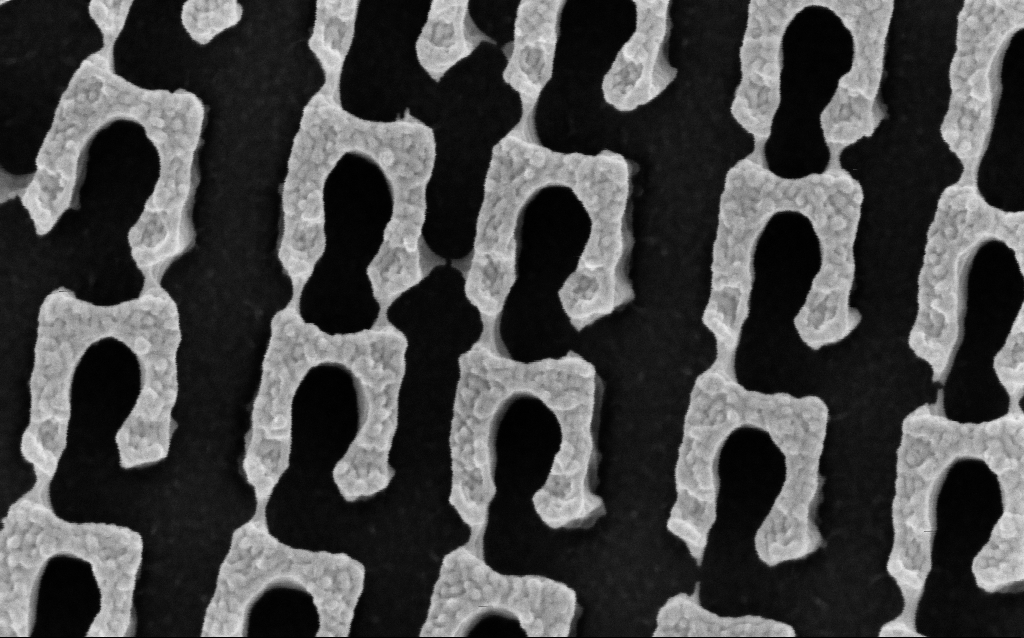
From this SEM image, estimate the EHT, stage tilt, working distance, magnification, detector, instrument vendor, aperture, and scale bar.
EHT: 3 kV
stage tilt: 0°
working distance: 4.6 mm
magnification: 175.6 K X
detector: InLens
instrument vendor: Zeiss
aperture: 30 µm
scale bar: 200 nm